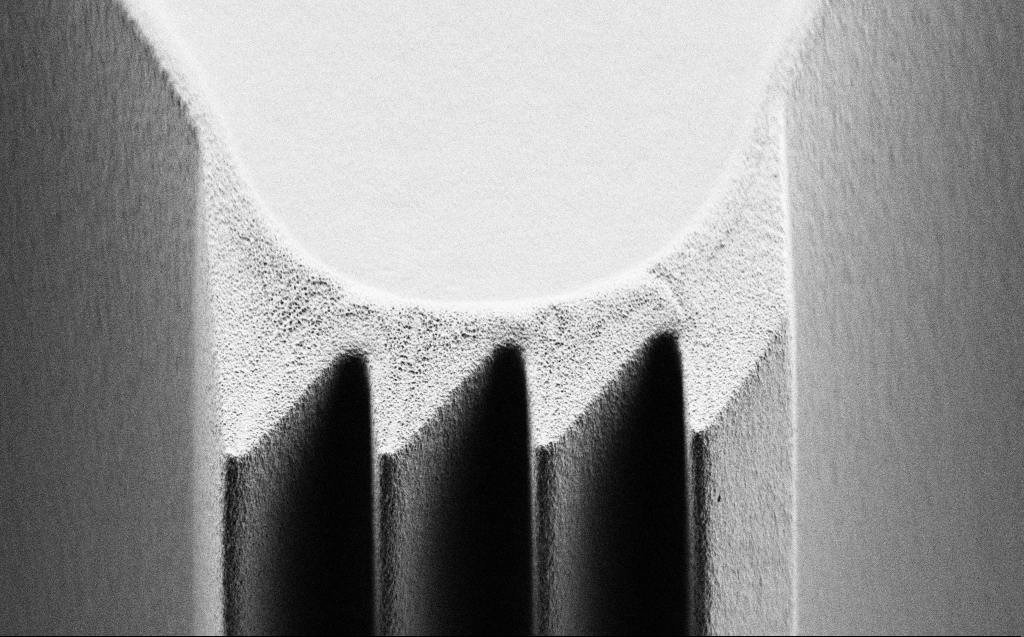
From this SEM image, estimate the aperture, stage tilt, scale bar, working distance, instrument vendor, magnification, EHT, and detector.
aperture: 30 µm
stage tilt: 45°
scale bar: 10000 nm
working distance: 4 mm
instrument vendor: Zeiss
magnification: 5.09 K X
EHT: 0.9 kV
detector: SE2